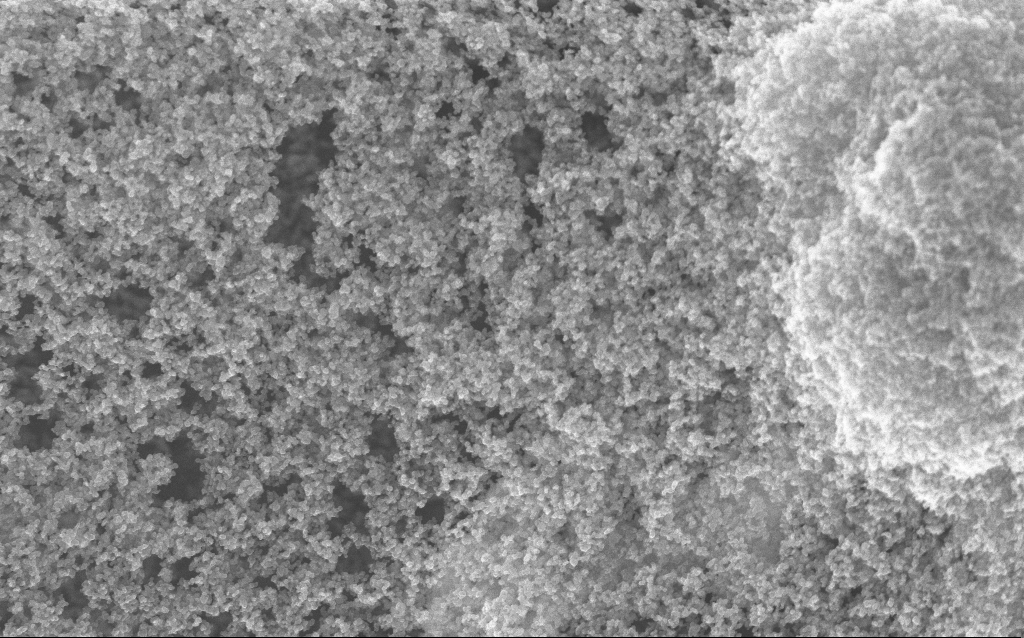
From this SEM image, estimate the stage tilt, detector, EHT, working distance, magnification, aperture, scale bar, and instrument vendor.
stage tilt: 0°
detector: InLens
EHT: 10 kV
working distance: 2.5 mm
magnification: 65.04 K X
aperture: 30 µm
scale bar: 1000 nm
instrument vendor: Zeiss